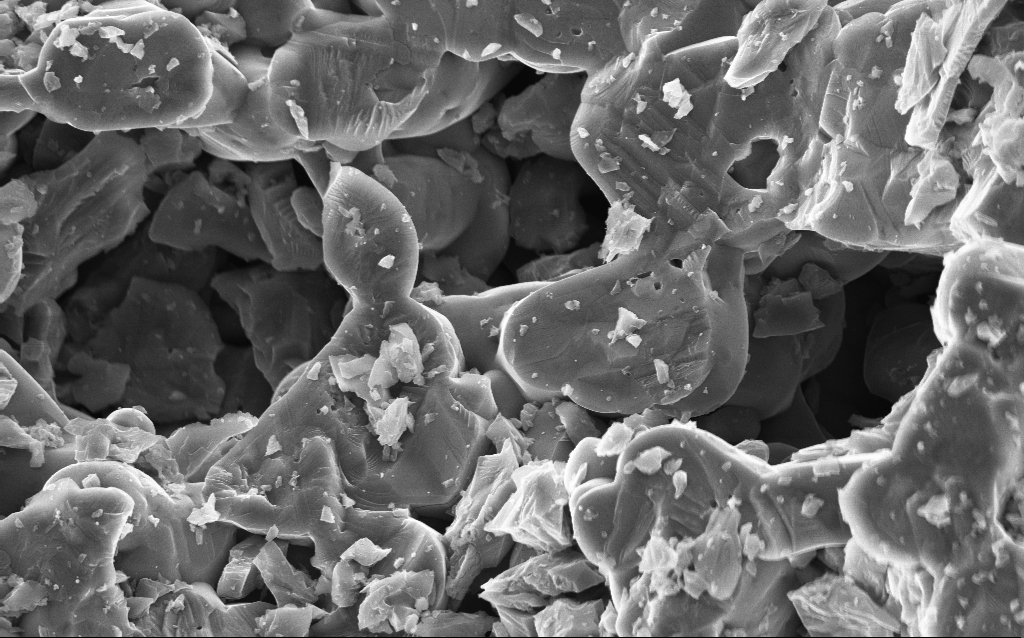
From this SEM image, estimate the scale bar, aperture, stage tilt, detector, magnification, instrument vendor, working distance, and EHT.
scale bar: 2000 nm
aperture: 30 µm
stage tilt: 0°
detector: InLens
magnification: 10 K X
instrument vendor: Zeiss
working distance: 3 mm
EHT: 10 kV